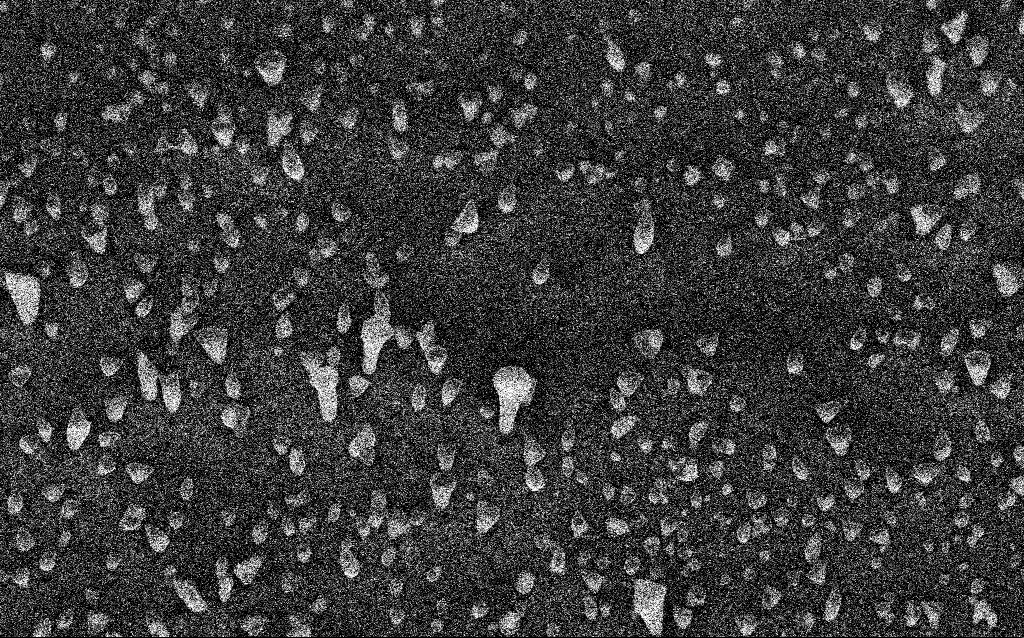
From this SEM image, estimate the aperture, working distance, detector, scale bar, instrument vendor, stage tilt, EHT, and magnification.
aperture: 30 µm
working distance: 7.3 mm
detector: InLens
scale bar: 1000 nm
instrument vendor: Zeiss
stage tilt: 45°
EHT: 5 kV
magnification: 50 K X